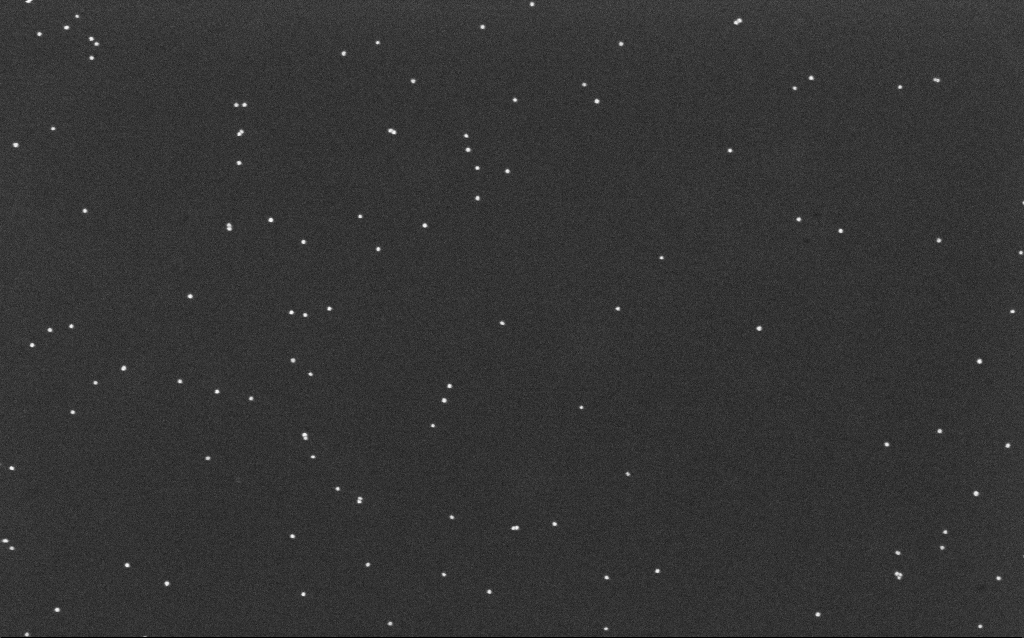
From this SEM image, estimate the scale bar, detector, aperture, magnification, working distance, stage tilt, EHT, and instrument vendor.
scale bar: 200 nm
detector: InLens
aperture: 30 µm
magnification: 100 K X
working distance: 6.4 mm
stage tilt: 0°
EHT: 10 kV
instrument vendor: Zeiss